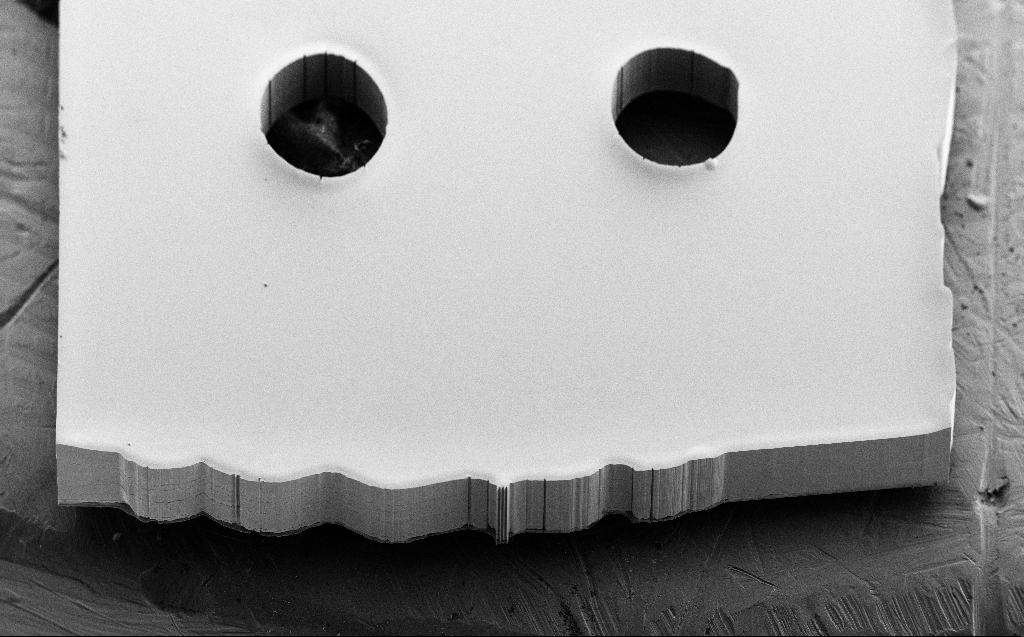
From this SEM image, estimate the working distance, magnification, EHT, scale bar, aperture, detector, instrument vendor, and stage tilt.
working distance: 4 mm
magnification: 0.109 K X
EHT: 10 kV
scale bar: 200000 nm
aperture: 30 µm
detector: SE2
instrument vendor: Zeiss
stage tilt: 45°